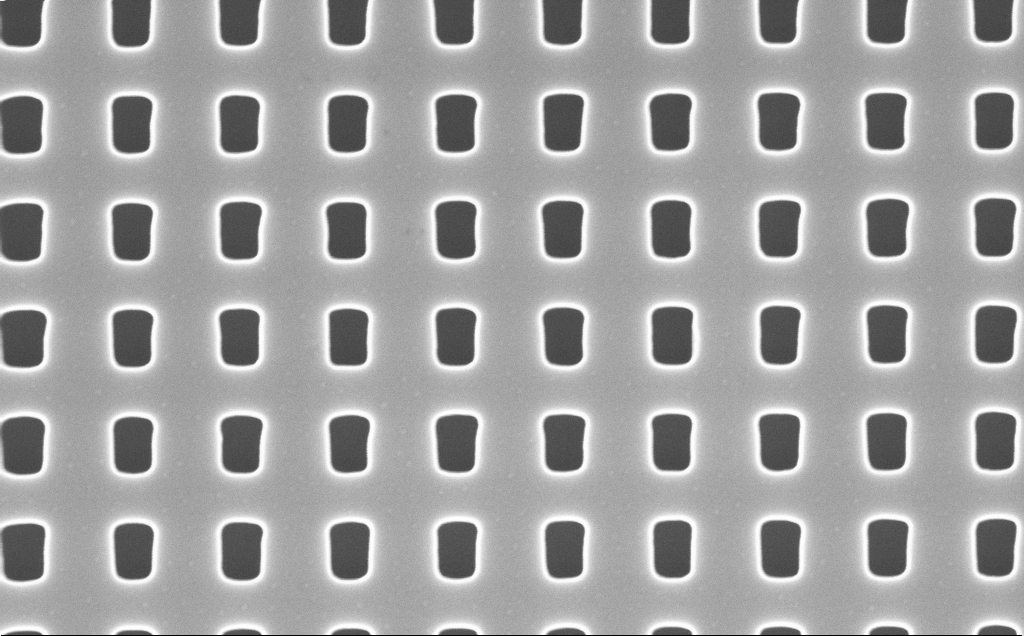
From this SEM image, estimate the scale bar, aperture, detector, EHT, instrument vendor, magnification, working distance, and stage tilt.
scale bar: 200 nm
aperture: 30 µm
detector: InLens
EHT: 10 kV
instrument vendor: Zeiss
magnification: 80 K X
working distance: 5 mm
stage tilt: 0°